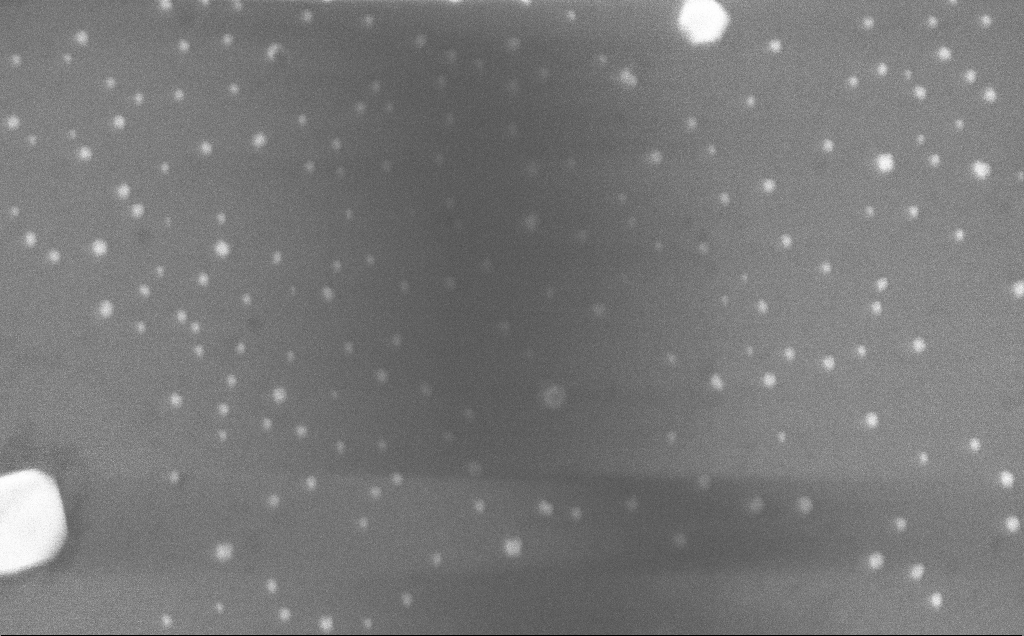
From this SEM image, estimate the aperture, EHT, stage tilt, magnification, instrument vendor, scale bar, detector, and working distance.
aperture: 30 µm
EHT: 10 kV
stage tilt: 0°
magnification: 103.39 K X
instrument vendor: Zeiss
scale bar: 200 nm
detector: InLens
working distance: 4 mm